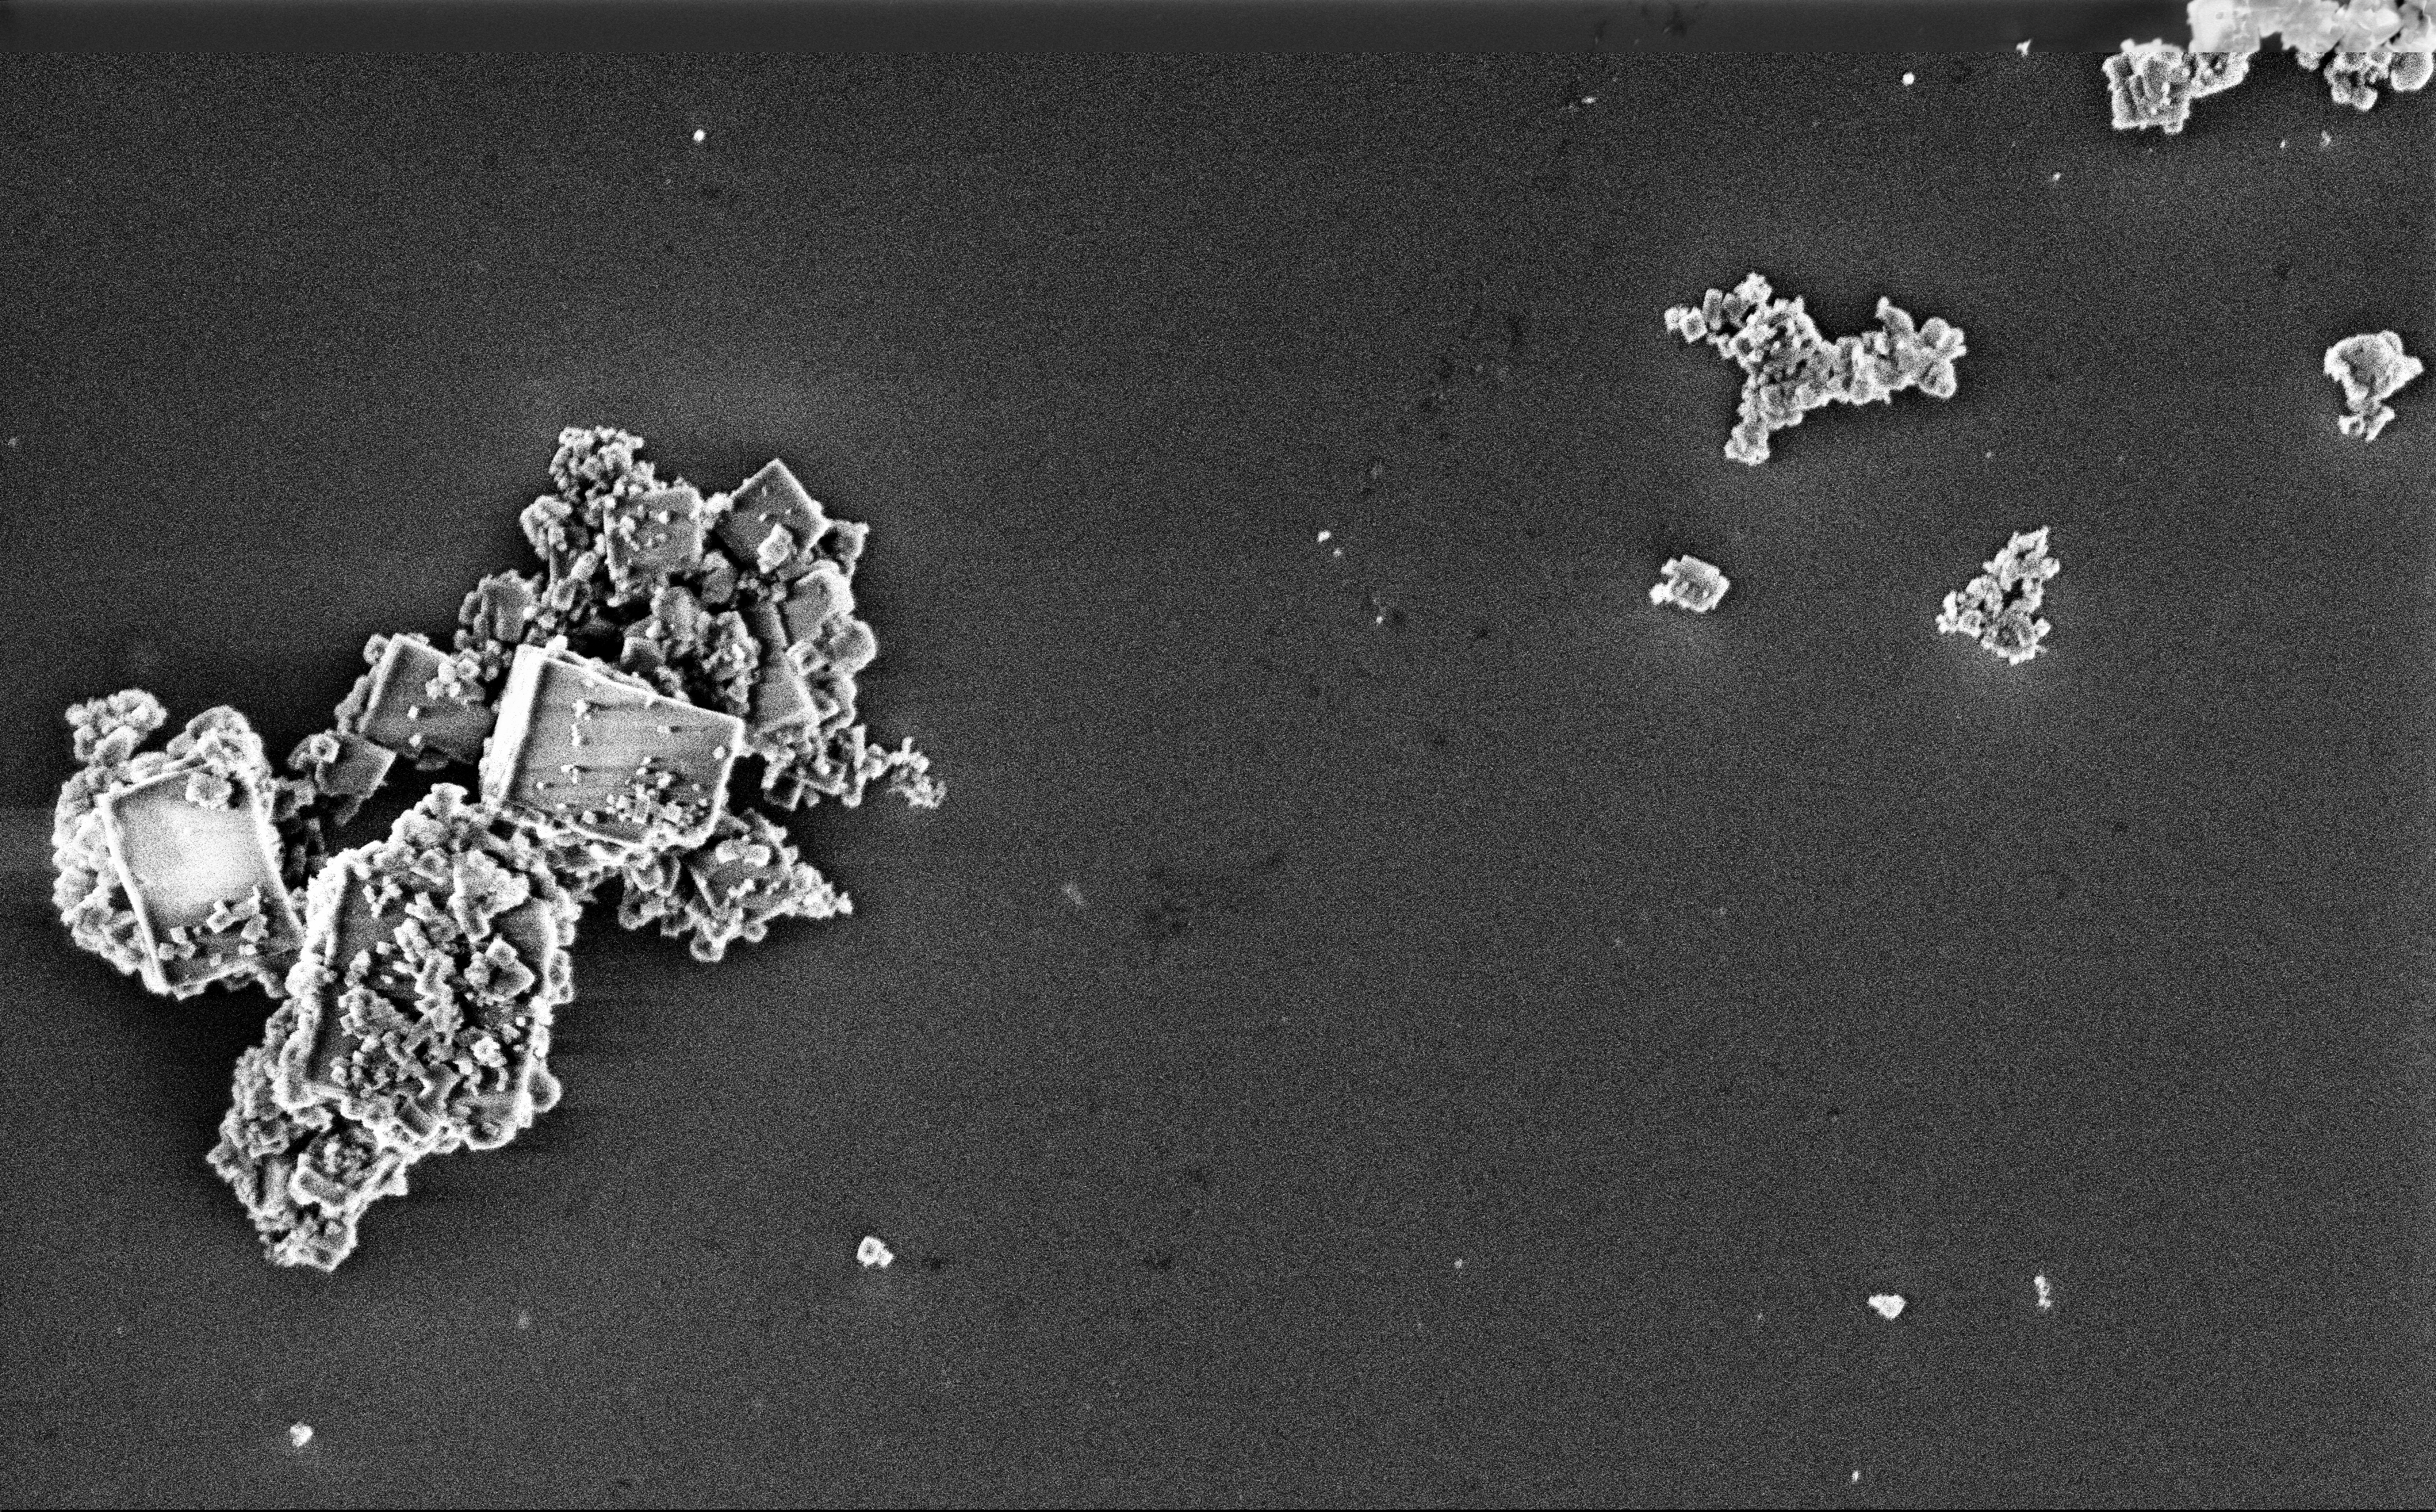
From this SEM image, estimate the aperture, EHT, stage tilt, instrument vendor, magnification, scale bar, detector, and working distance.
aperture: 30 µm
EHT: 3 kV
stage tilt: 0°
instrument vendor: Zeiss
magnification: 13.53 K X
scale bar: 2000 nm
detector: InLens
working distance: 3 mm